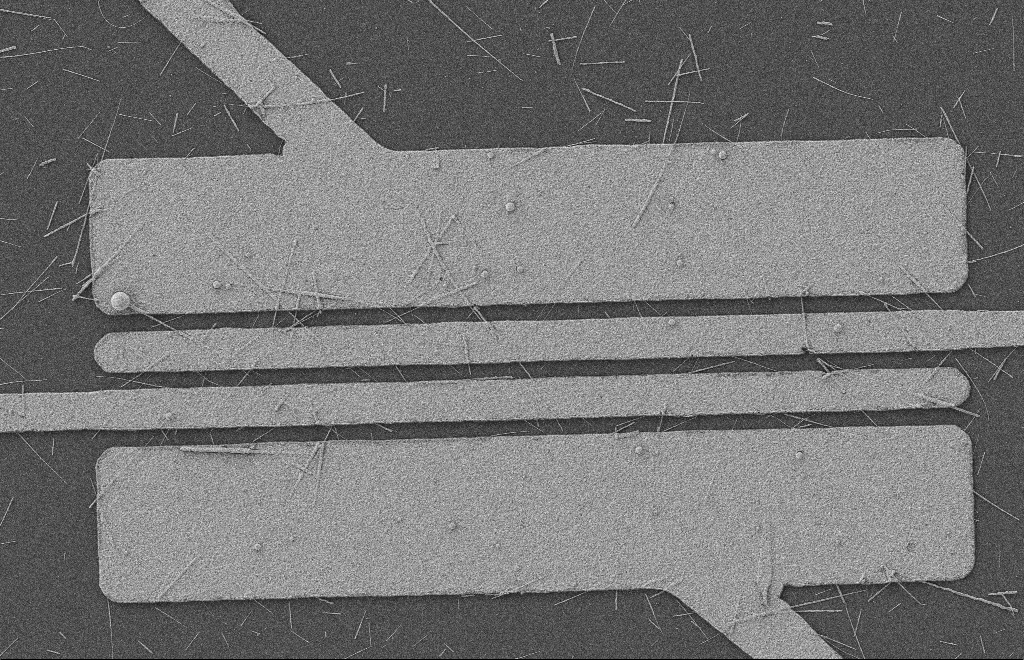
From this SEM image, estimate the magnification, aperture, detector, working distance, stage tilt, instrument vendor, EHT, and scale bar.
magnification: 5.28 K X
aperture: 20 µm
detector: SE2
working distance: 8 mm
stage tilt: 0°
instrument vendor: Zeiss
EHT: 2 kV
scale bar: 2000 nm